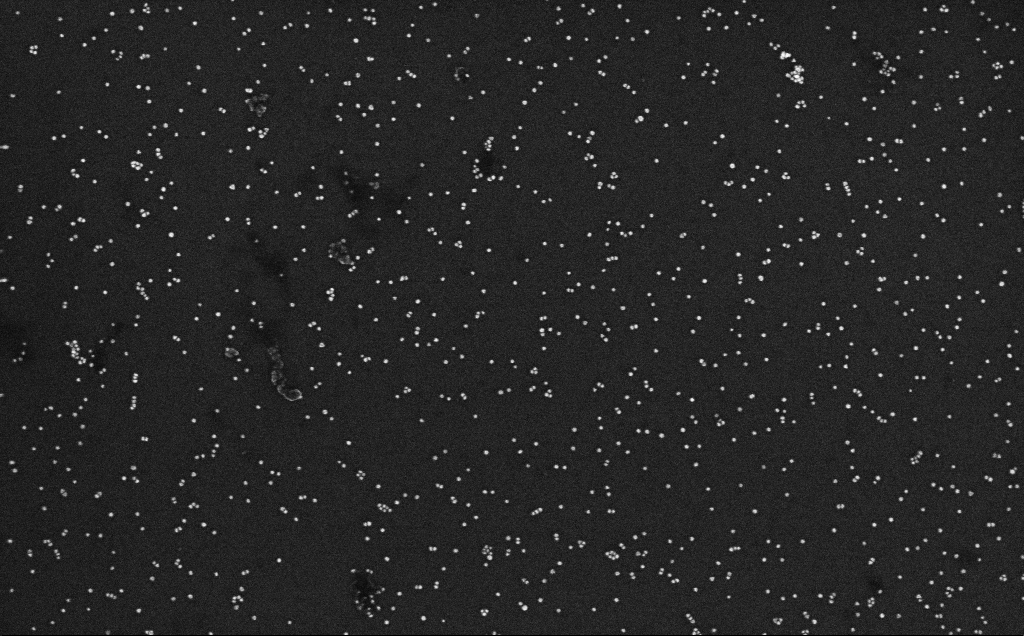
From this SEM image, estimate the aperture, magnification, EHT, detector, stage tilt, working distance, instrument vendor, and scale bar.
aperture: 30 µm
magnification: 100 K X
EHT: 10 kV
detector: InLens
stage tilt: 0°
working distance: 3.3 mm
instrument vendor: Zeiss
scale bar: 200 nm